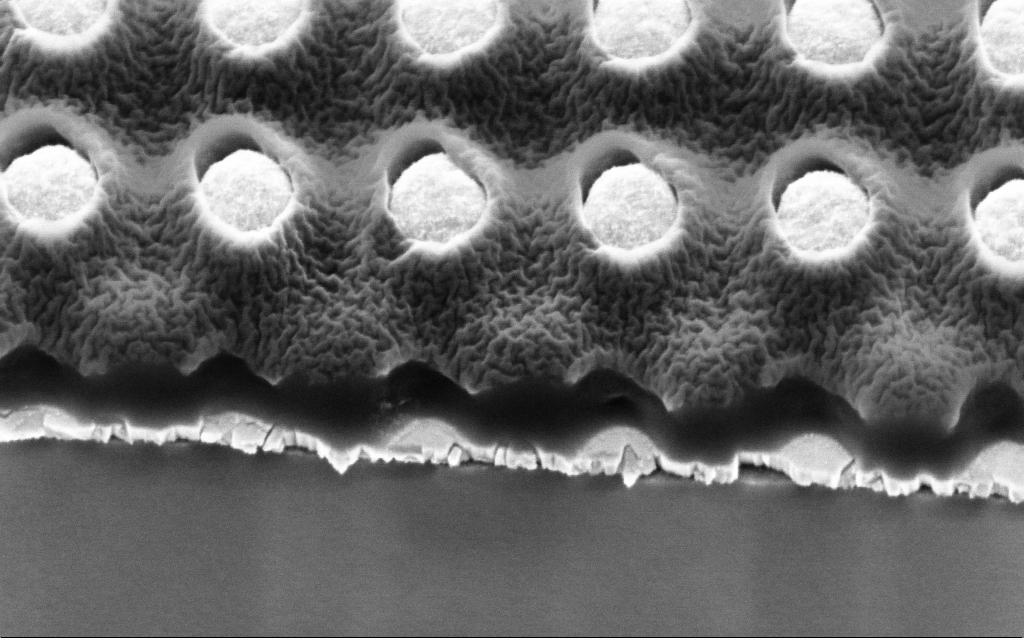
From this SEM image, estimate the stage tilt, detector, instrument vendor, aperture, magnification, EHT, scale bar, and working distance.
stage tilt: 45°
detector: InLens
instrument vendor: Zeiss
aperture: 30 µm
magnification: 142.87 K X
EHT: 2 kV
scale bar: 200 nm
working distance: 3.5 mm